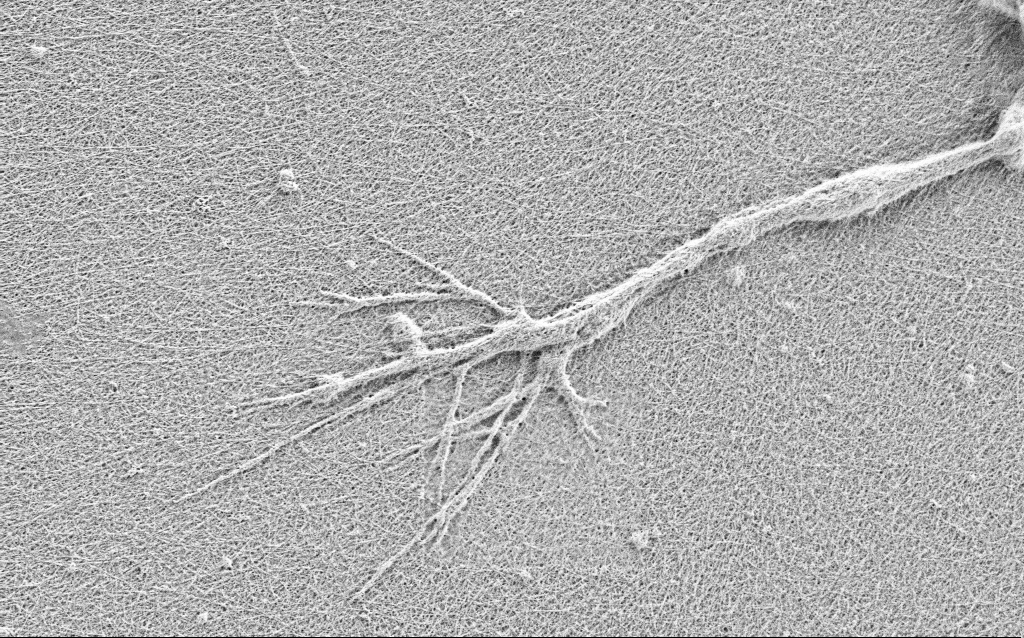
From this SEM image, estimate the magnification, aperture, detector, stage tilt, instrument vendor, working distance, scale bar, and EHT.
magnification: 10 K X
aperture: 30 µm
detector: SE2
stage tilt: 0°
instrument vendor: Zeiss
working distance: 3 mm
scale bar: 2000 nm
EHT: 0.9 kV